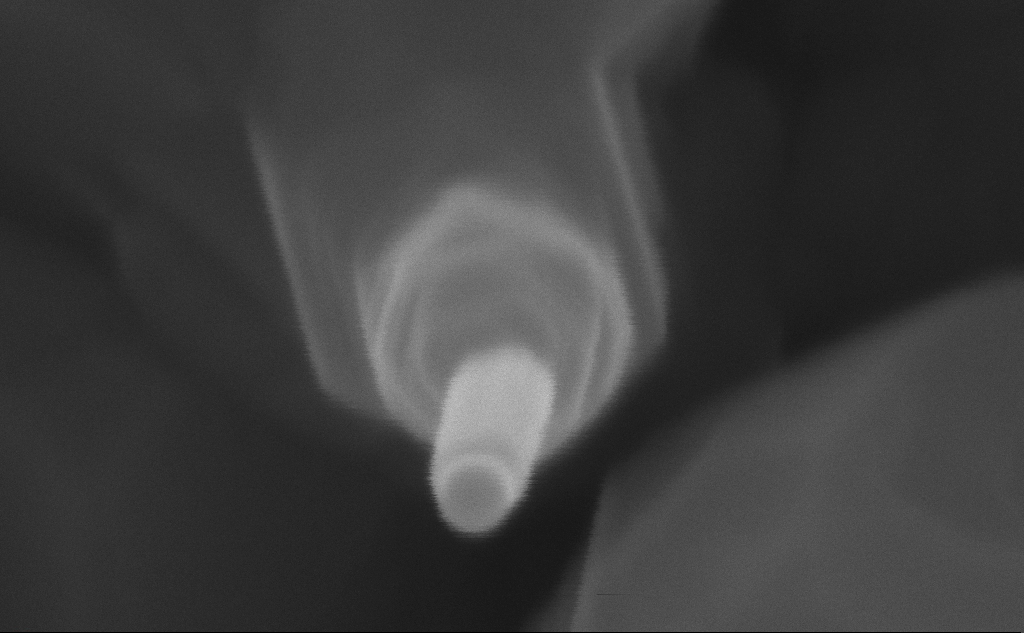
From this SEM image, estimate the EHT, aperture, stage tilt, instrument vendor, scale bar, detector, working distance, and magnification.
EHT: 10 kV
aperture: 30 µm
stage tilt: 0°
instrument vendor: Zeiss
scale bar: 20 nm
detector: InLens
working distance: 7 mm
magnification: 782.01 K X